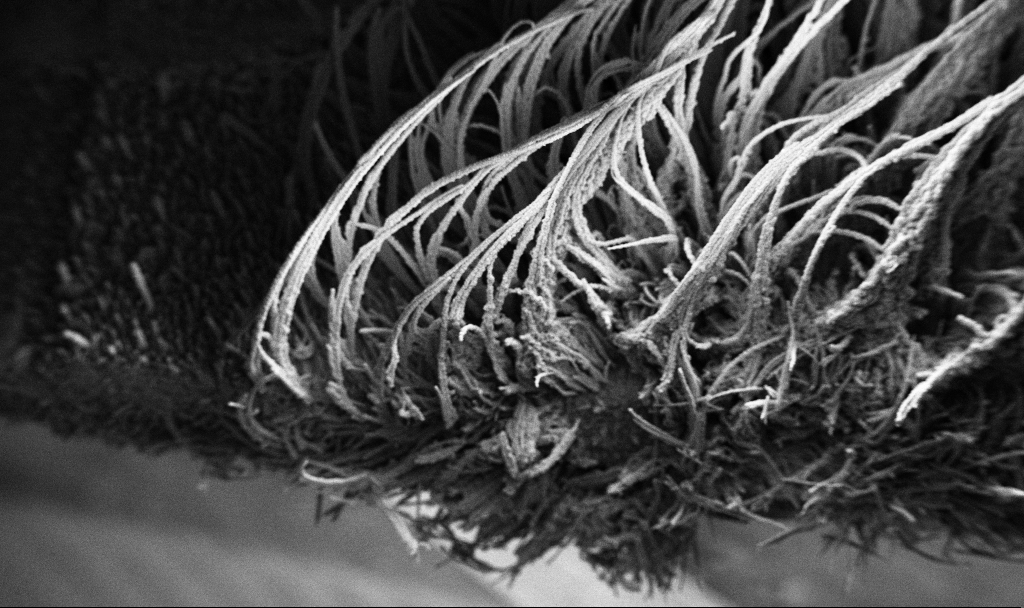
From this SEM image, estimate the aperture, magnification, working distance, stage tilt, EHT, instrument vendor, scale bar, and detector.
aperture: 30 µm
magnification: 0.15 K X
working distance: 7.5 mm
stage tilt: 37.7°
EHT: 3 kV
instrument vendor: Zeiss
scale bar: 100000 nm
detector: InLens